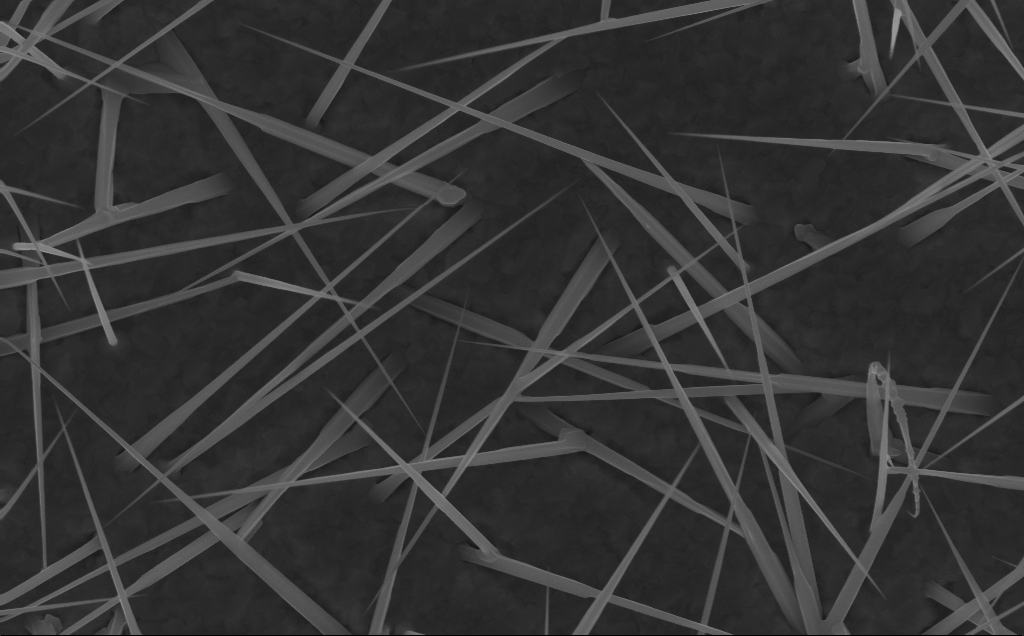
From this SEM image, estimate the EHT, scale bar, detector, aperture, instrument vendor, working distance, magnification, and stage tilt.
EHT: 10 kV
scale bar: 1000 nm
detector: InLens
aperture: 30 µm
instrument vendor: Zeiss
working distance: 6 mm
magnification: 40 K X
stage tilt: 0°